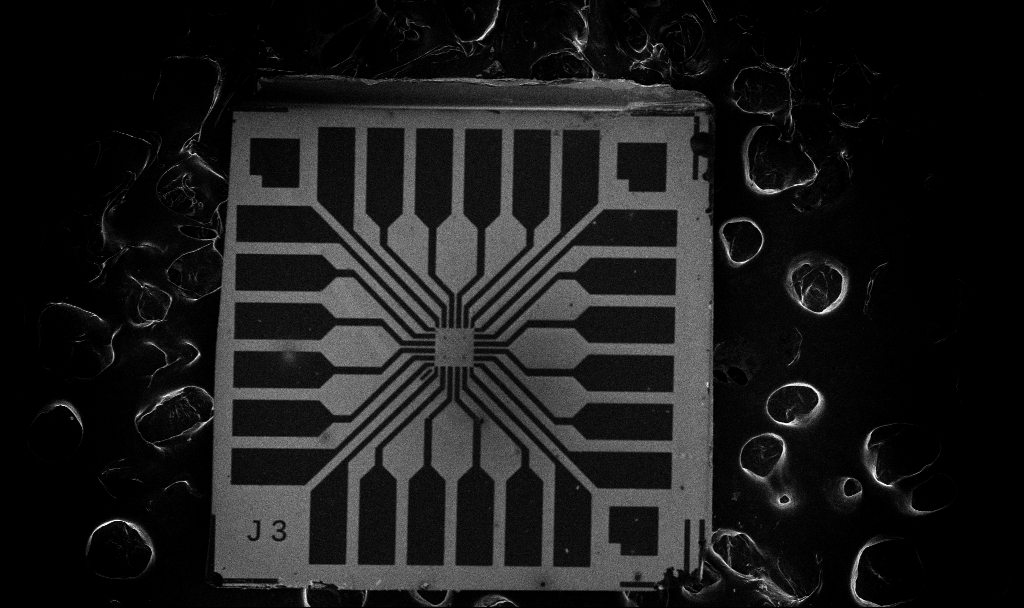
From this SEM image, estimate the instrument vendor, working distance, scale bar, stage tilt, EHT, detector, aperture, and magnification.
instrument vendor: Zeiss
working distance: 7.7 mm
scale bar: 200000 nm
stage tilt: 0°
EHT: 10 kV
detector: InLens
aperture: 30 µm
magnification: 0.087 K X